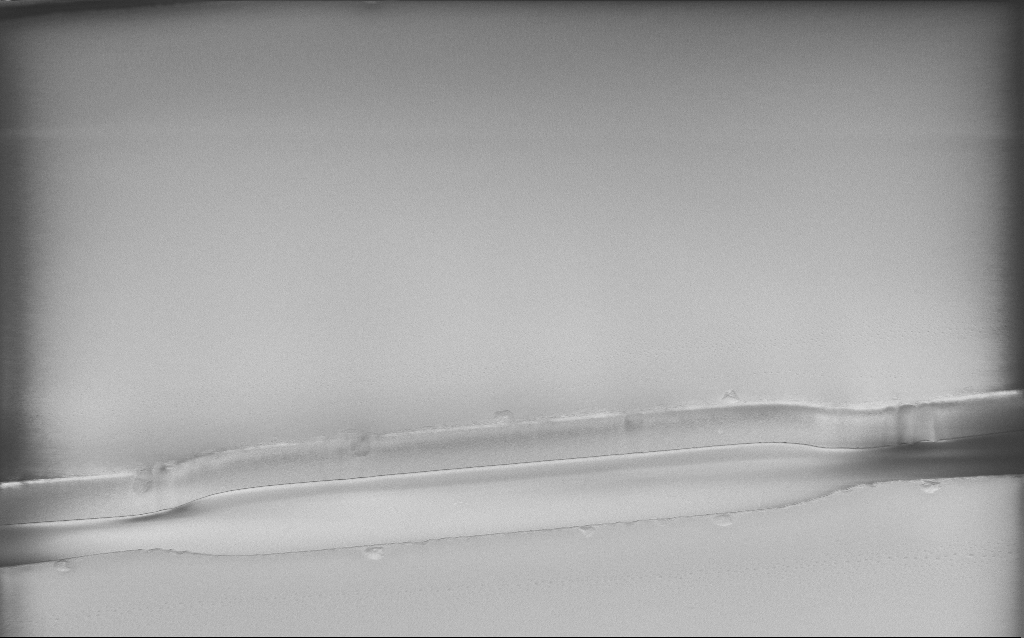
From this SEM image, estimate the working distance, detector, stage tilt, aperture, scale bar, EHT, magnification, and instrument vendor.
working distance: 6 mm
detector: InLens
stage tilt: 45°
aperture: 30 µm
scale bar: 20000 nm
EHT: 1.2 kV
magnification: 2.6 K X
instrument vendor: Zeiss